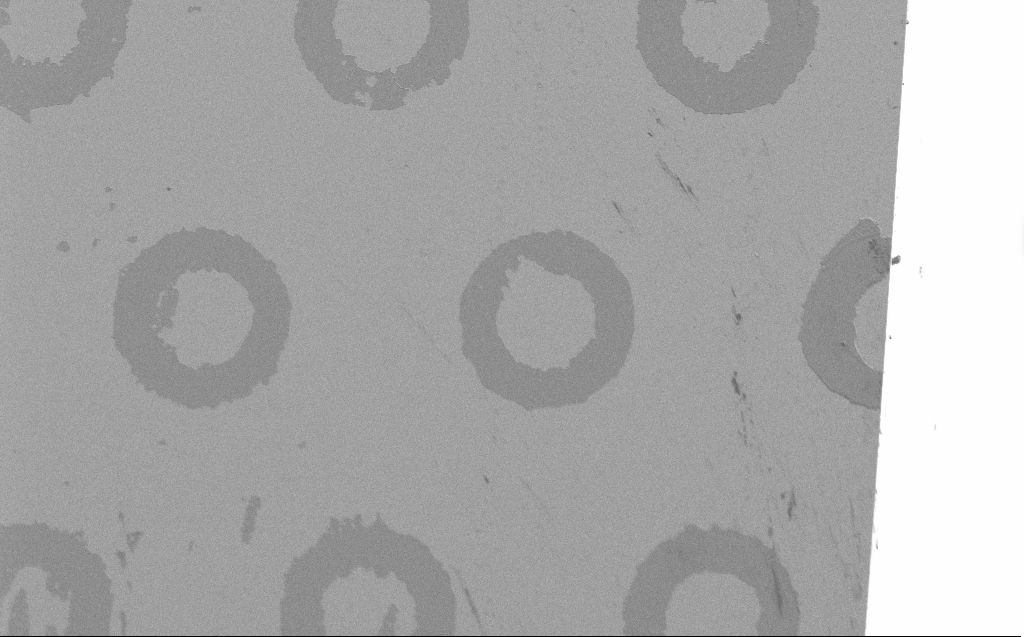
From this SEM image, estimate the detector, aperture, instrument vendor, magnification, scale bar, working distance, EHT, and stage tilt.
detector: SE2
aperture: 30 µm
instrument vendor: Zeiss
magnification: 1.29 K X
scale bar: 20000 nm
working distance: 3 mm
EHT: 2 kV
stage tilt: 26.3°